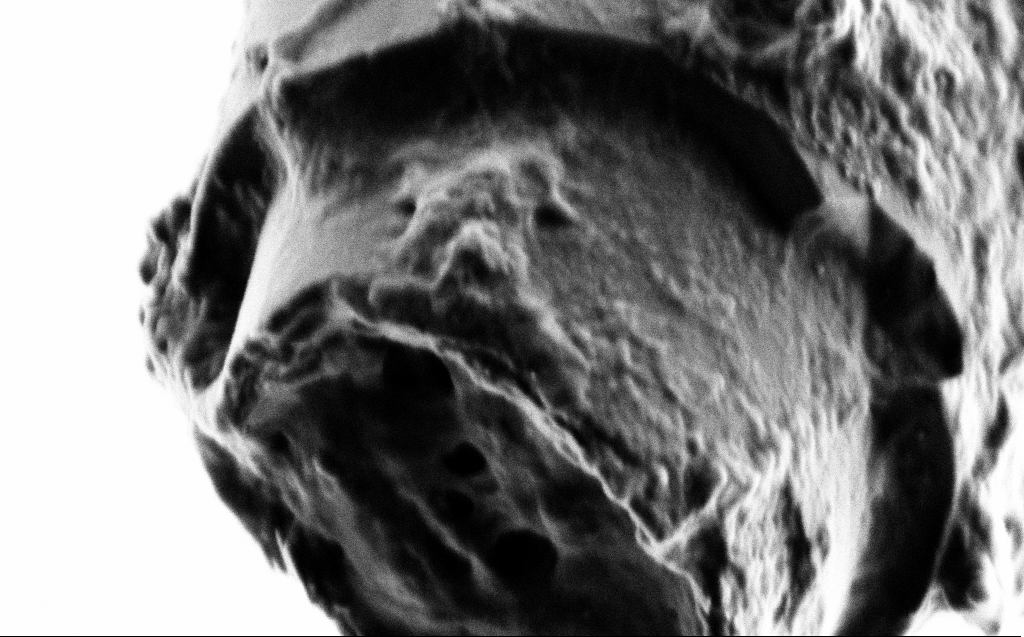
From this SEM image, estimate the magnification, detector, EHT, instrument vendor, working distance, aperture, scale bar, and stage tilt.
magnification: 92.36 K X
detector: SE2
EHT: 1 kV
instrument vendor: Zeiss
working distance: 4 mm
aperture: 30 µm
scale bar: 200 nm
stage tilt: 45°